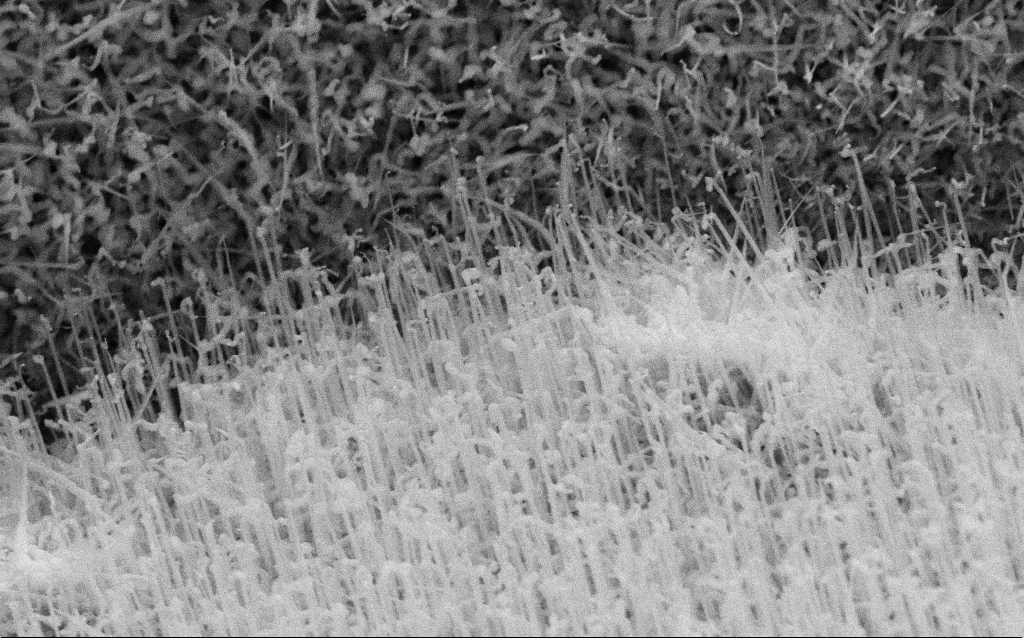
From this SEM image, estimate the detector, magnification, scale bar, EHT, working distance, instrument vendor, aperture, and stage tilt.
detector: SE2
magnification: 45.95 K X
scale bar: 1000 nm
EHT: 10 kV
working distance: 7.6 mm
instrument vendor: Zeiss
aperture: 30 µm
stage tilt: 0°